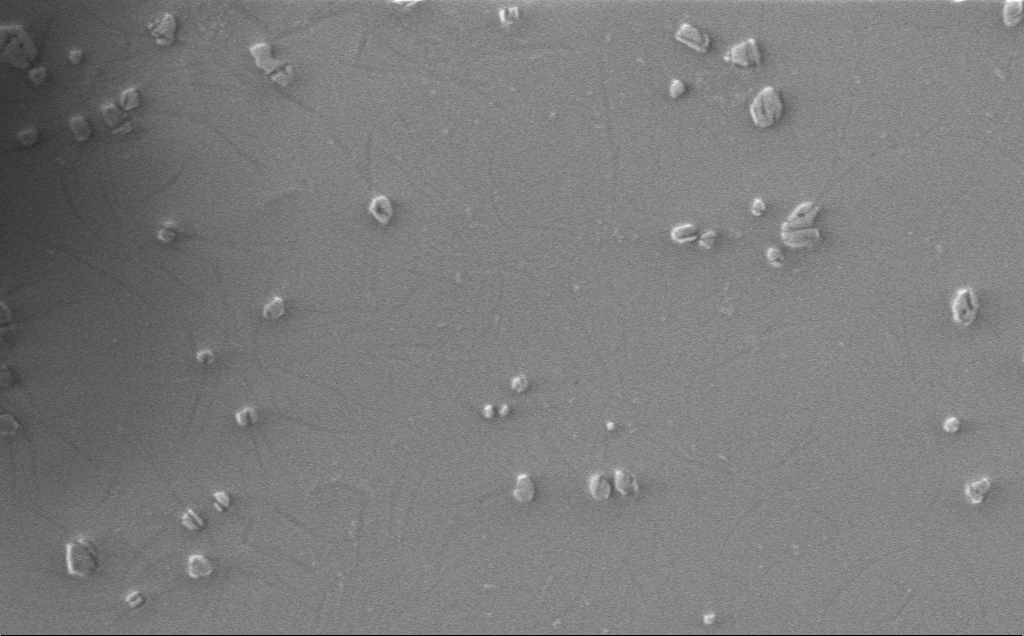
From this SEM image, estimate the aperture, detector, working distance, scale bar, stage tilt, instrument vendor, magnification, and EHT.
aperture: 30 µm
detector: InLens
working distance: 4 mm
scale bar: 2000 nm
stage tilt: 0°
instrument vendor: Zeiss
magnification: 9.1 K X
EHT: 1 kV